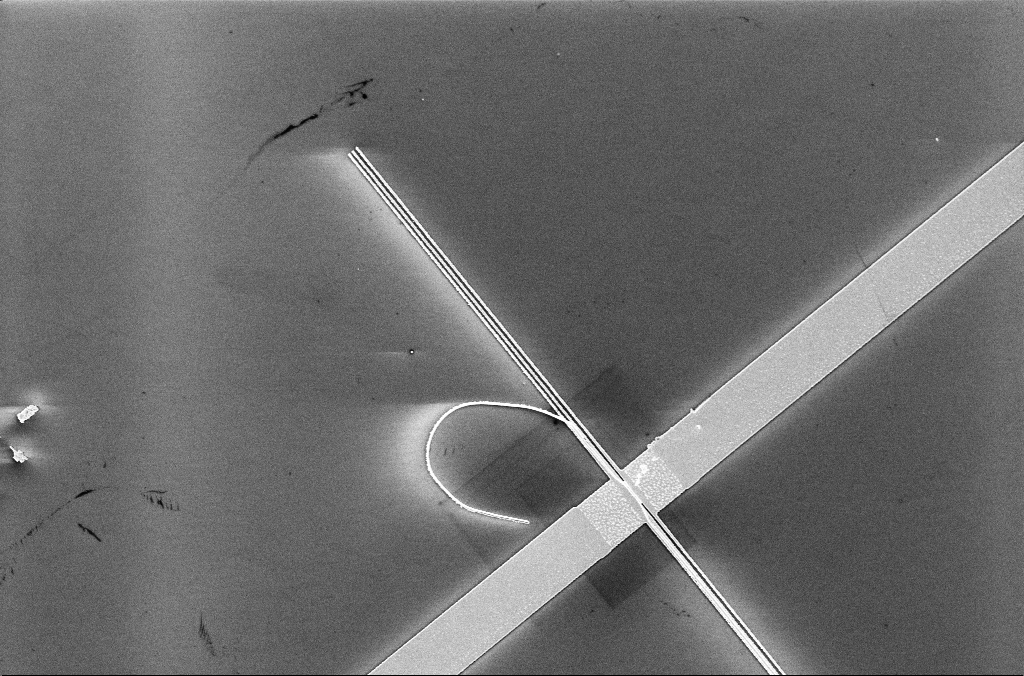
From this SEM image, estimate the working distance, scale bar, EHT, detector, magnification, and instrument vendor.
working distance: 3.3 mm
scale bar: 10000 nm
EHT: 5 kV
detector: InLens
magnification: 4.17 K X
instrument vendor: Zeiss